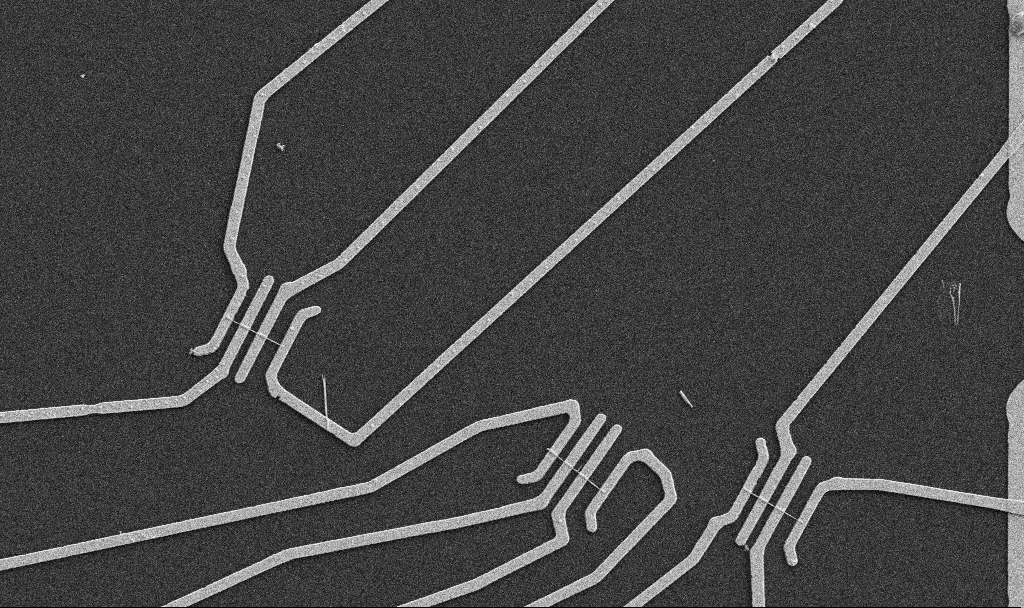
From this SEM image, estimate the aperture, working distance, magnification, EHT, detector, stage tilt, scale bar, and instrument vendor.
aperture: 30 µm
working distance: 10.7 mm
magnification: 5 K X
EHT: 5 kV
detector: SE2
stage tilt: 0°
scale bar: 10000 nm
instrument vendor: Zeiss